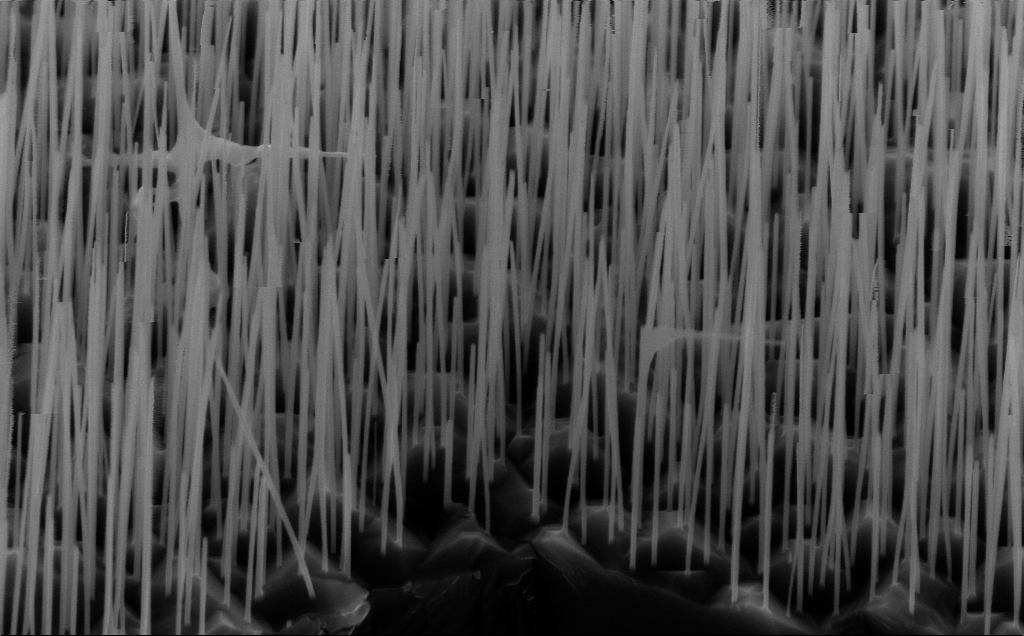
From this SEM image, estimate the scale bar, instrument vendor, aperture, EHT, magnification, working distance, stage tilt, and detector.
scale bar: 1000 nm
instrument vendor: Zeiss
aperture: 30 µm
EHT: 10 kV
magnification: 45.16 K X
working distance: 5 mm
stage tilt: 45°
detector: InLens